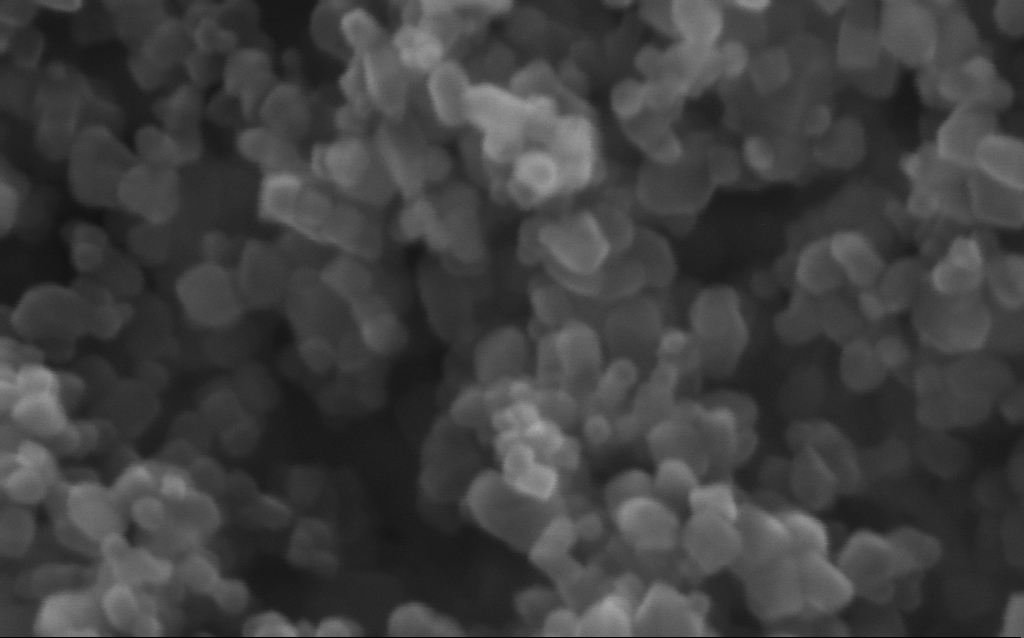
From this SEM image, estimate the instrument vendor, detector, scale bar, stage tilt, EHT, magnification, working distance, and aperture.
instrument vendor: Zeiss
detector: InLens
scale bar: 100 nm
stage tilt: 0°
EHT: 10 kV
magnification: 716 K X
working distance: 2.6 mm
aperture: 30 µm